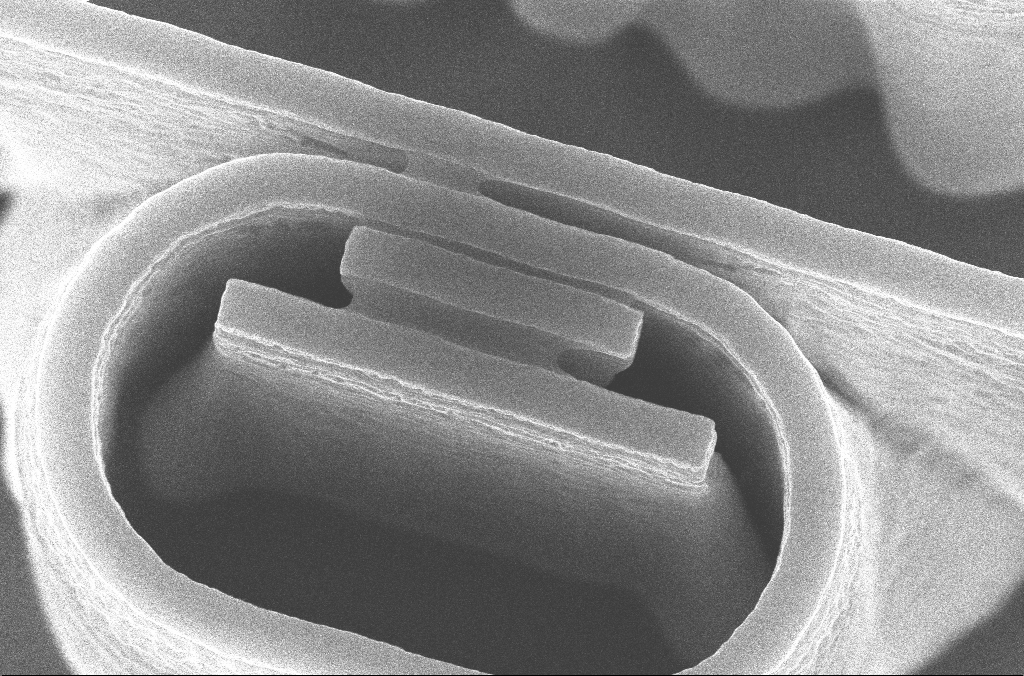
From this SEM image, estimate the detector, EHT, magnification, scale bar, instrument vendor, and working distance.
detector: InLens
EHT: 10 kV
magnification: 37.29 K X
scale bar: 1000 nm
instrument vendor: Zeiss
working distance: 7.3 mm